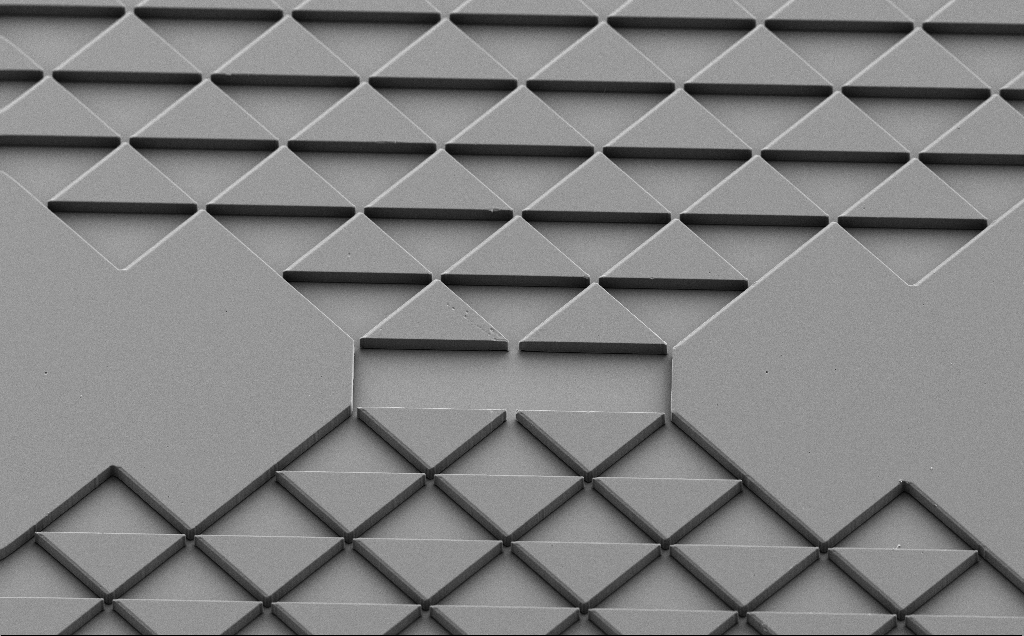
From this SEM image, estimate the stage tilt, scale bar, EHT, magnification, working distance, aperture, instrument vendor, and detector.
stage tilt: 40°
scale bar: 100000 nm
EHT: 5 kV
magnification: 0.619 K X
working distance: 9 mm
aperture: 30 µm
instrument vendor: Zeiss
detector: SE2